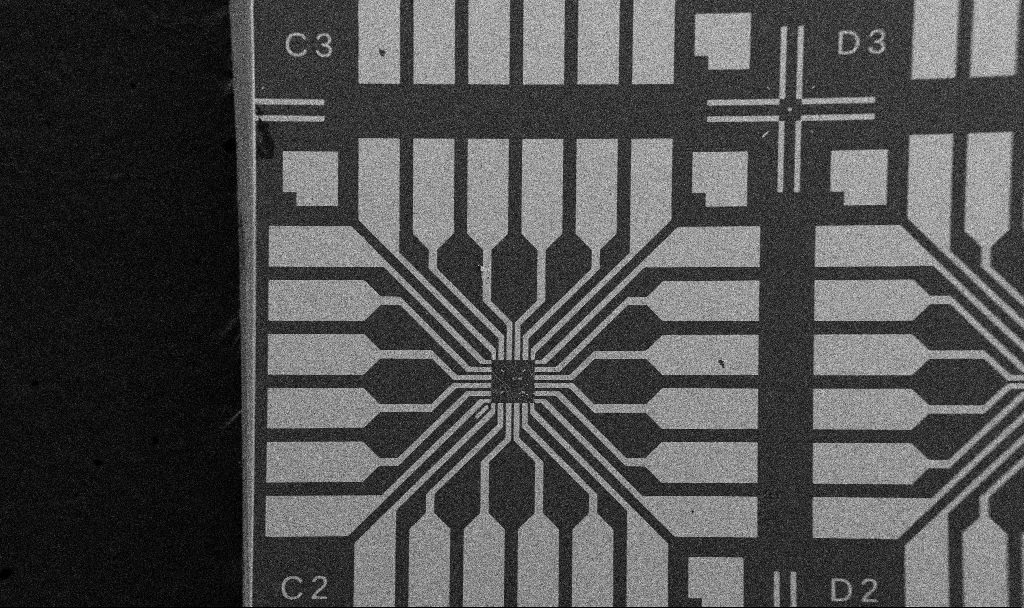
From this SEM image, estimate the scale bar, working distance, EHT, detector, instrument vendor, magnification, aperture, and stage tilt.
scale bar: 200000 nm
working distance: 10.7 mm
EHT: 5 kV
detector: SE2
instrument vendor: Zeiss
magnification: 0.1 K X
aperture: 30 µm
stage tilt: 0°